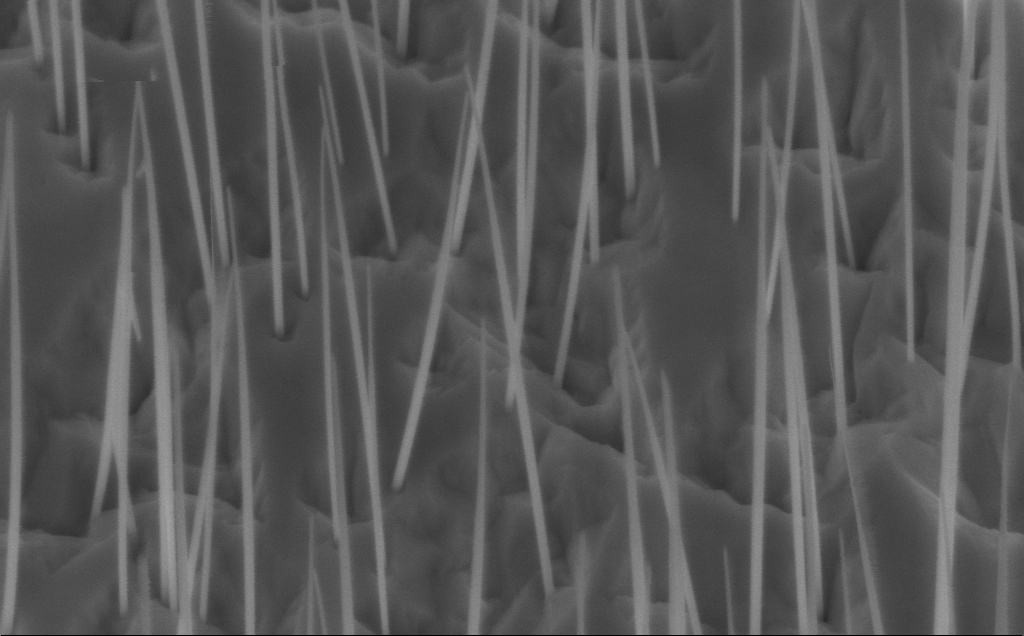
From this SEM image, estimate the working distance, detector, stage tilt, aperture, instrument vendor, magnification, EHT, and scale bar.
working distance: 6 mm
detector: InLens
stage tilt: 45°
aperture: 30 µm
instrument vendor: Zeiss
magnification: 80 K X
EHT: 5 kV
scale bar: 200 nm